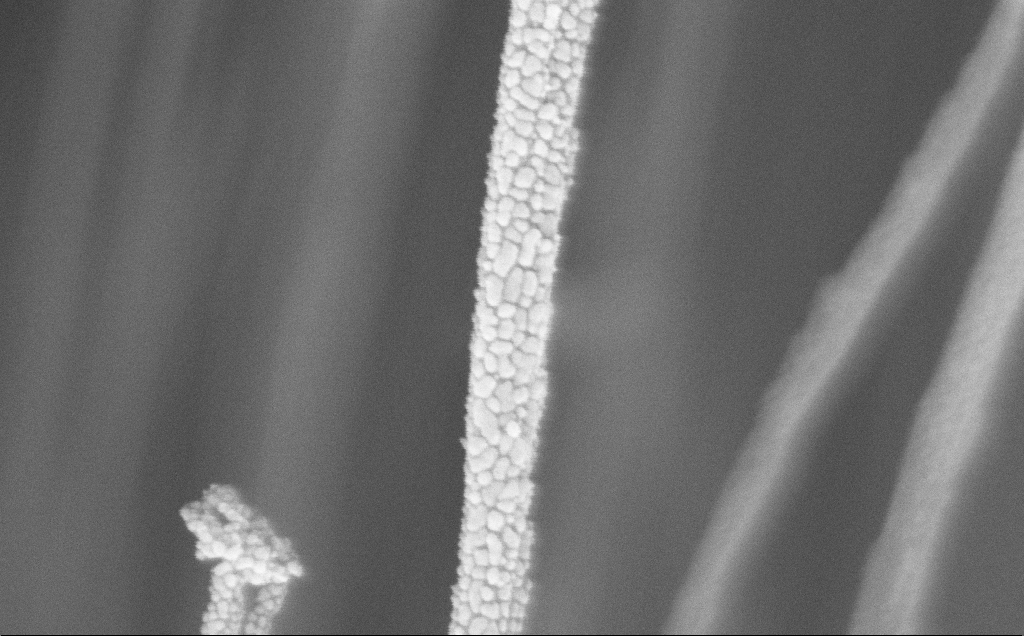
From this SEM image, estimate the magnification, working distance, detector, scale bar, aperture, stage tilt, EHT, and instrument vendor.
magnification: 163.96 K X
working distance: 11 mm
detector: InLens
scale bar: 200 nm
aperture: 30 µm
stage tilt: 0°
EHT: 5 kV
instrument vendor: Zeiss